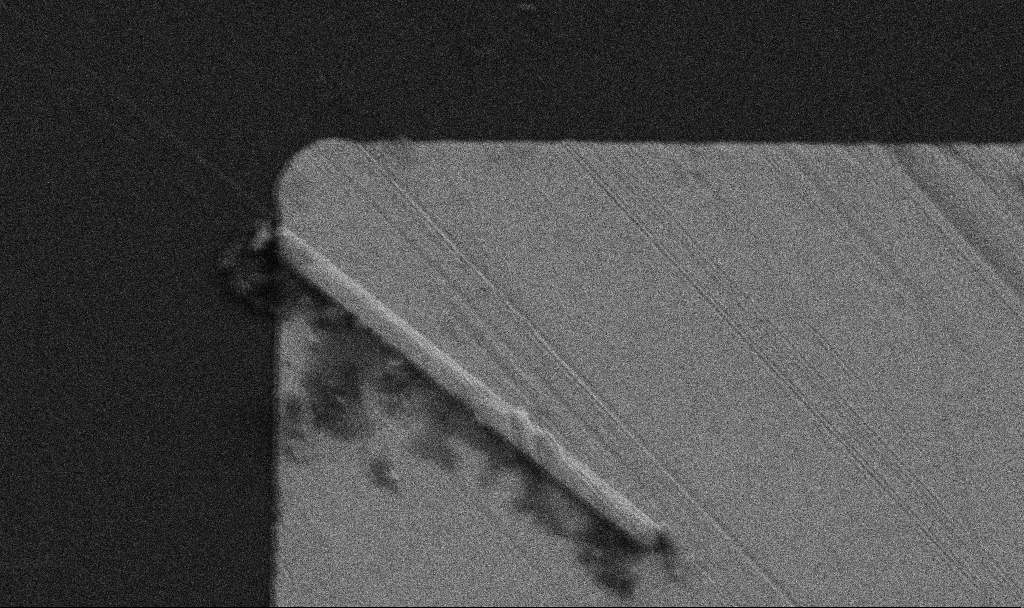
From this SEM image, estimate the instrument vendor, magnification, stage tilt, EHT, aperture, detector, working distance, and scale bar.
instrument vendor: Zeiss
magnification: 13 K X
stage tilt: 0°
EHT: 5 kV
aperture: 30 µm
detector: SE2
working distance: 8.9 mm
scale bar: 1000 nm